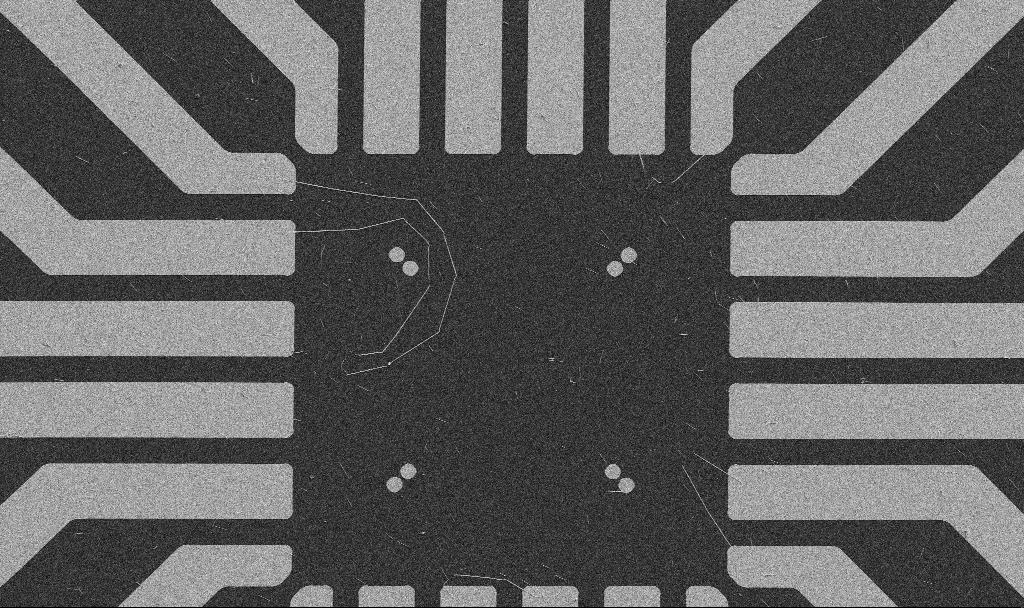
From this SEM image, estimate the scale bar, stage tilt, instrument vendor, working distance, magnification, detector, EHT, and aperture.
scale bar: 20000 nm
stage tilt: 0°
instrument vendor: Zeiss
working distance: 10.7 mm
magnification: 1 K X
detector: SE2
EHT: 5 kV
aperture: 30 µm